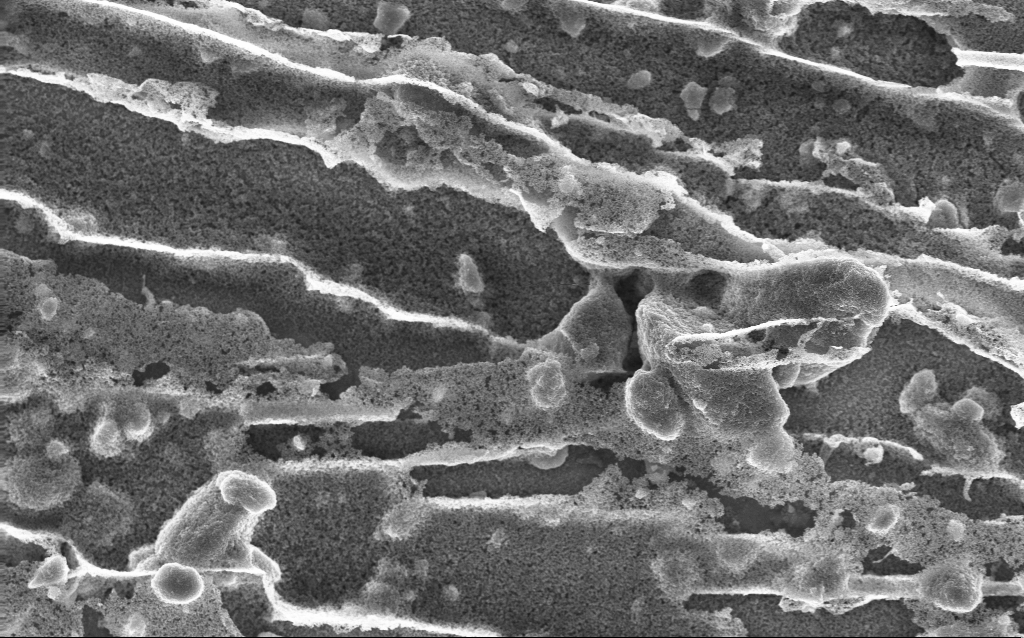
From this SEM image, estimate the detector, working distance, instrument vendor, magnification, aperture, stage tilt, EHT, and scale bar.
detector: InLens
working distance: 2.5 mm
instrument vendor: Zeiss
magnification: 5.83 K X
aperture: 30 µm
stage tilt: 0°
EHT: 10 kV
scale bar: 10000 nm